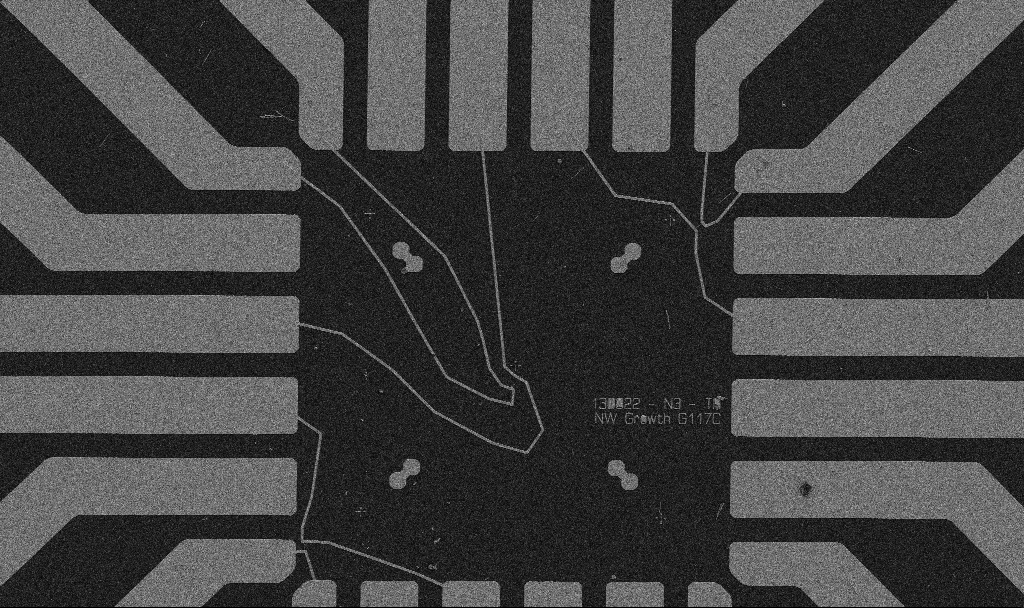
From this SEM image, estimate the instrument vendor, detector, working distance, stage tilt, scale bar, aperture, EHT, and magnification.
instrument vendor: Zeiss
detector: SE2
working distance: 10.7 mm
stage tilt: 0°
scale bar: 20000 nm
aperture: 30 µm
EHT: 5 kV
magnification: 1 K X